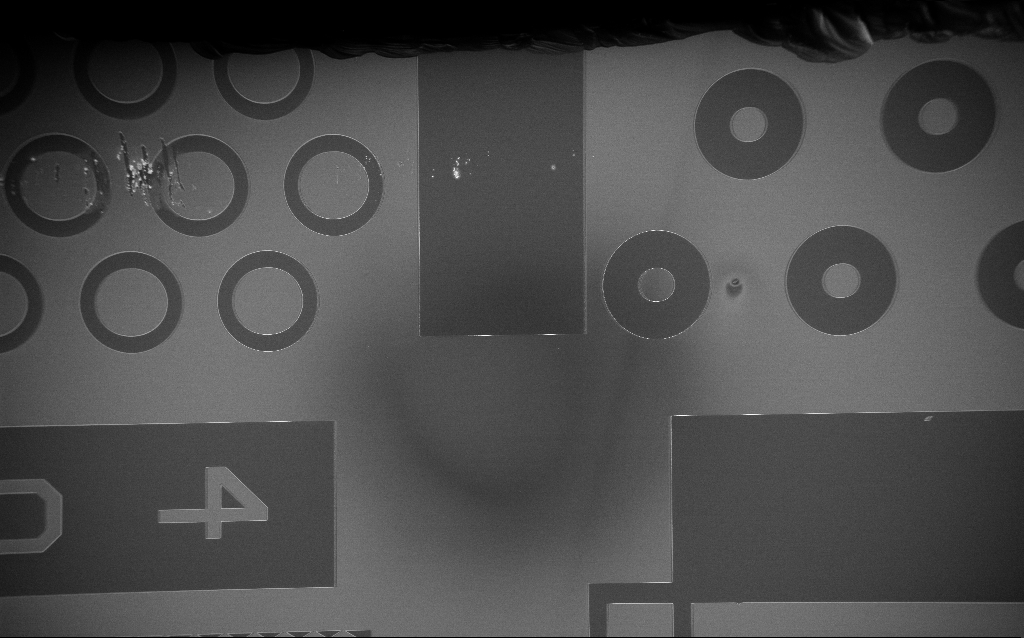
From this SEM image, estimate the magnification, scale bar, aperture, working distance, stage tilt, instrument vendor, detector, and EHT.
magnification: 0.156 K X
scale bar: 200000 nm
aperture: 30 µm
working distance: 5 mm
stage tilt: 0°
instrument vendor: Zeiss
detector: InLens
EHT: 3 kV